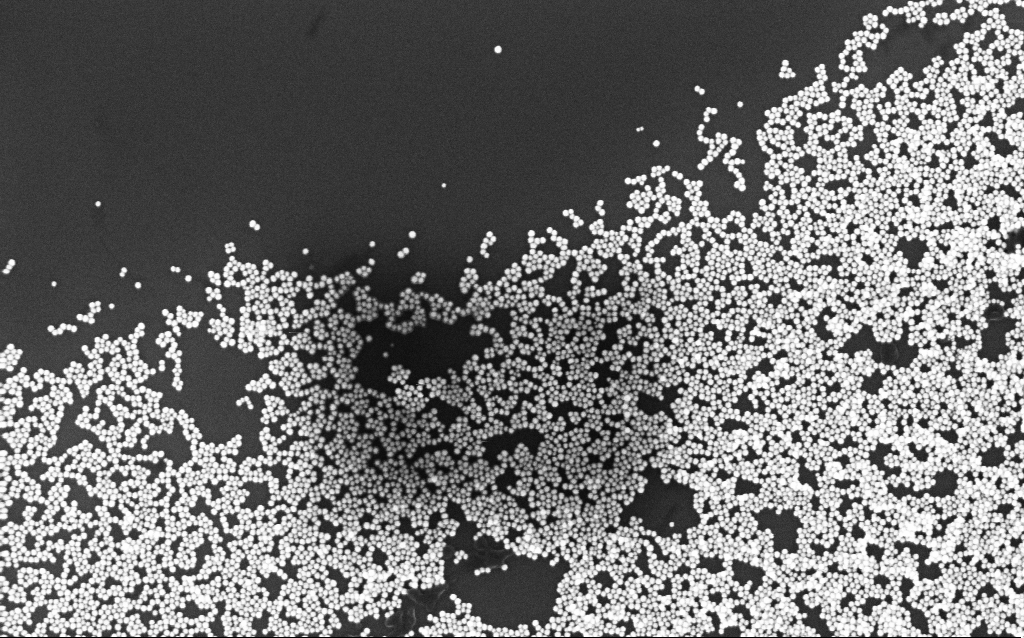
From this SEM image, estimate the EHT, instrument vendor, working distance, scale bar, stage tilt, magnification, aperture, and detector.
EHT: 5 kV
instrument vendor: Zeiss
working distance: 3.3 mm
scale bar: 200 nm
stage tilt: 0°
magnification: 100 K X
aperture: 30 µm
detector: InLens